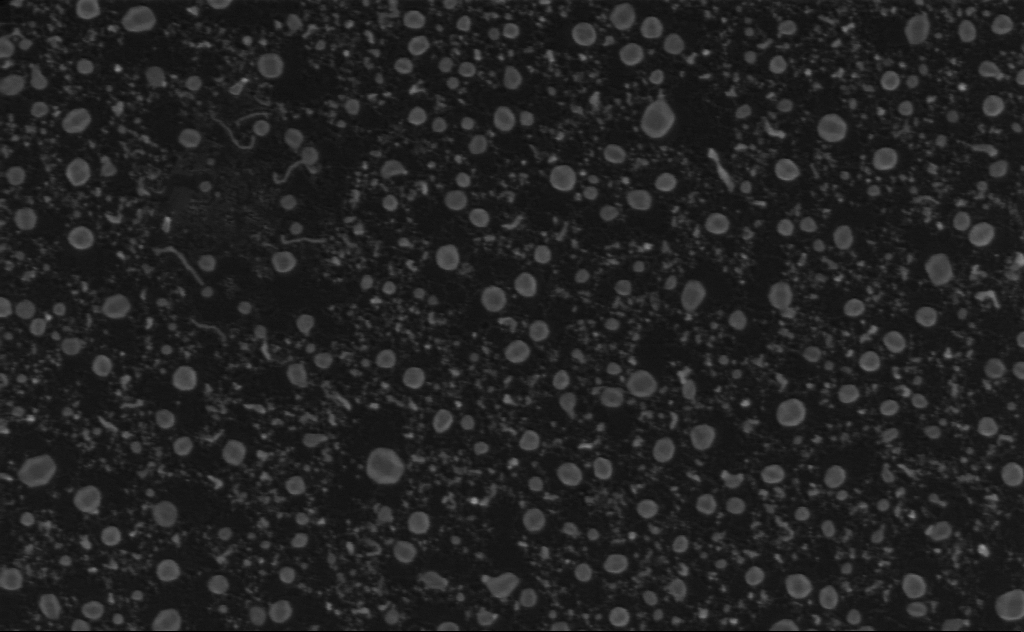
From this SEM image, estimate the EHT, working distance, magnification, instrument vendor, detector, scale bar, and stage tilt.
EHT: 1 kV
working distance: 4 mm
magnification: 80 K X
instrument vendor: Zeiss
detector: InLens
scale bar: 200 nm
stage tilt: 0°